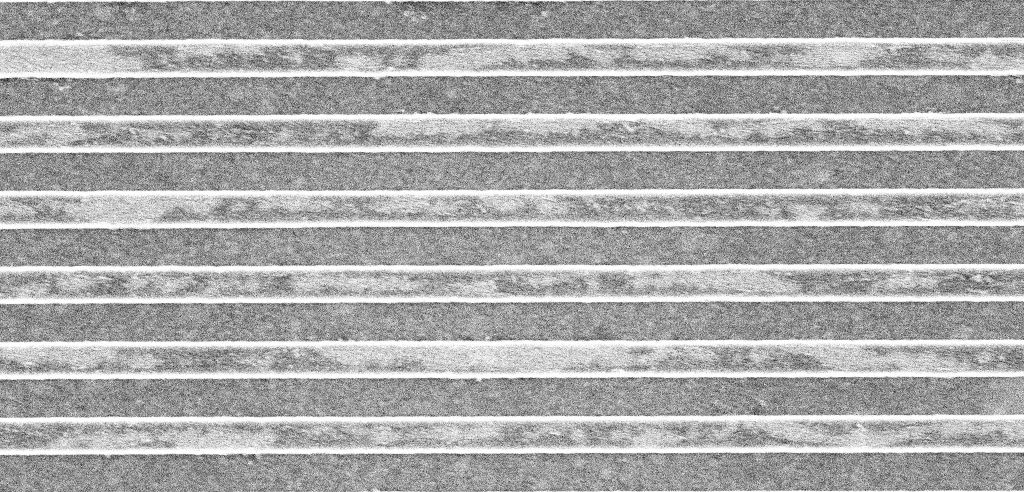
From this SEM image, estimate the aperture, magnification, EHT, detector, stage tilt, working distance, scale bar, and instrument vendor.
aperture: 30 µm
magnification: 46.5 K X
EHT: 5 kV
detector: InLens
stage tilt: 0°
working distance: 3.1 mm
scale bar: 1000 nm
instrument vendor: Zeiss